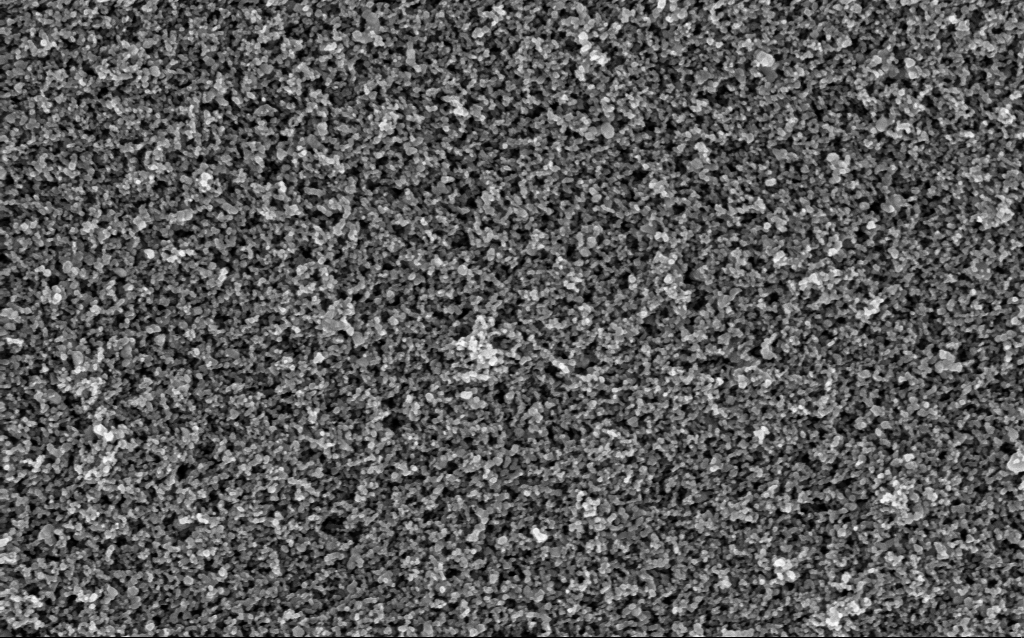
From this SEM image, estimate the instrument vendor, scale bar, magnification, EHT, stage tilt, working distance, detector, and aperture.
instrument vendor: Zeiss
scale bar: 1000 nm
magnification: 65.04 K X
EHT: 5 kV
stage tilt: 0°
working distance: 4.9 mm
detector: InLens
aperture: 30 µm